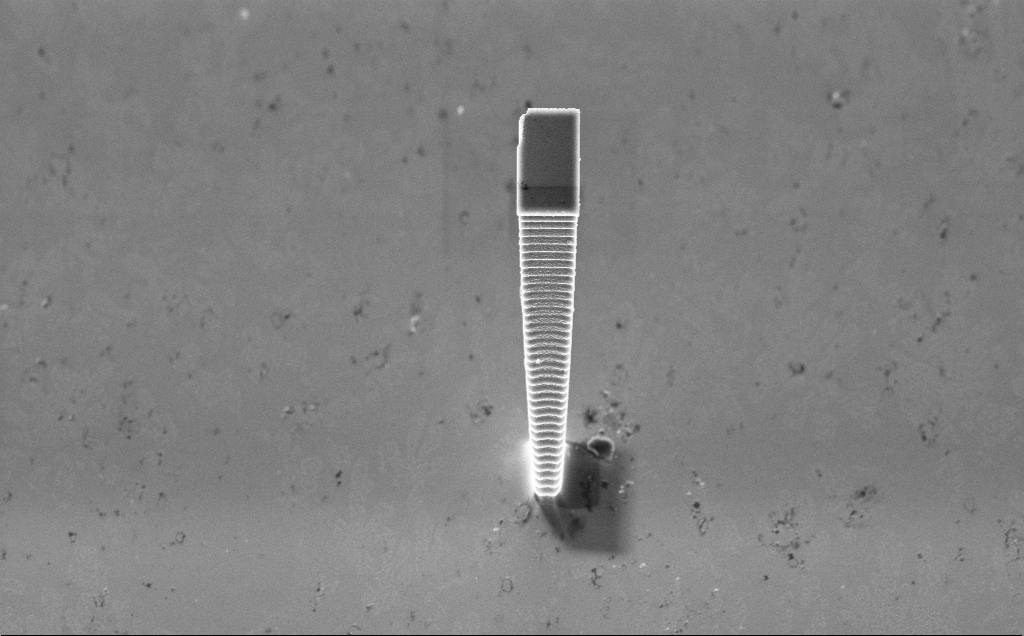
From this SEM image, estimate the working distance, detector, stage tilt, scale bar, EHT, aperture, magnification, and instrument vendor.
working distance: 7 mm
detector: InLens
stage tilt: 45°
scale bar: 2000 nm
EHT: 5 kV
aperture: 30 µm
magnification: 7.96 K X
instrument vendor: Zeiss